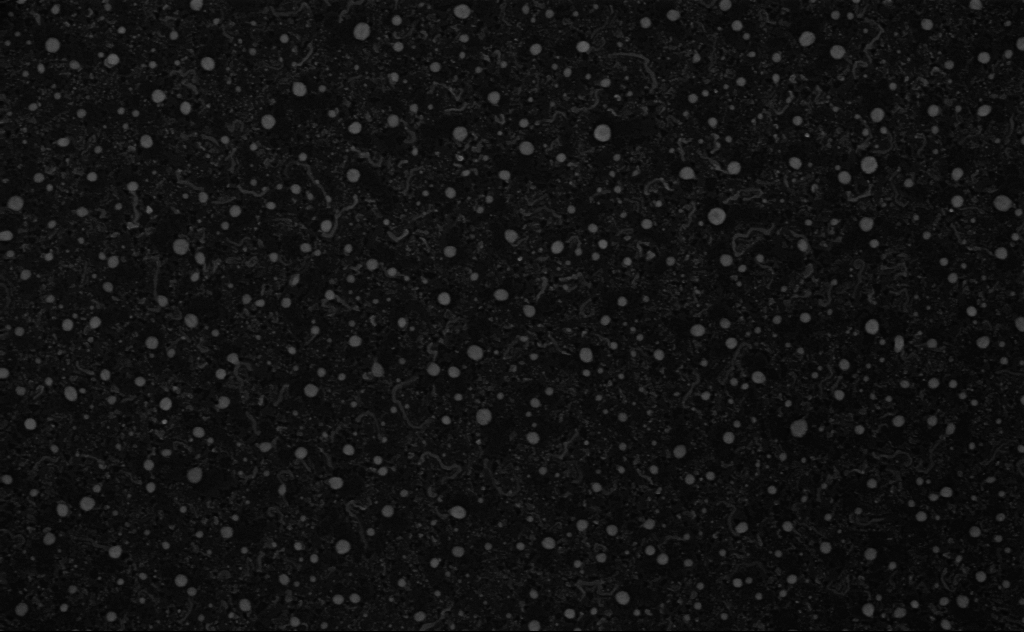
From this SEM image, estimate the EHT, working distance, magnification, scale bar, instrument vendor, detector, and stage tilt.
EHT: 3 kV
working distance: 4 mm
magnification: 80 K X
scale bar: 200 nm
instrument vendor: Zeiss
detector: InLens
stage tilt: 0°